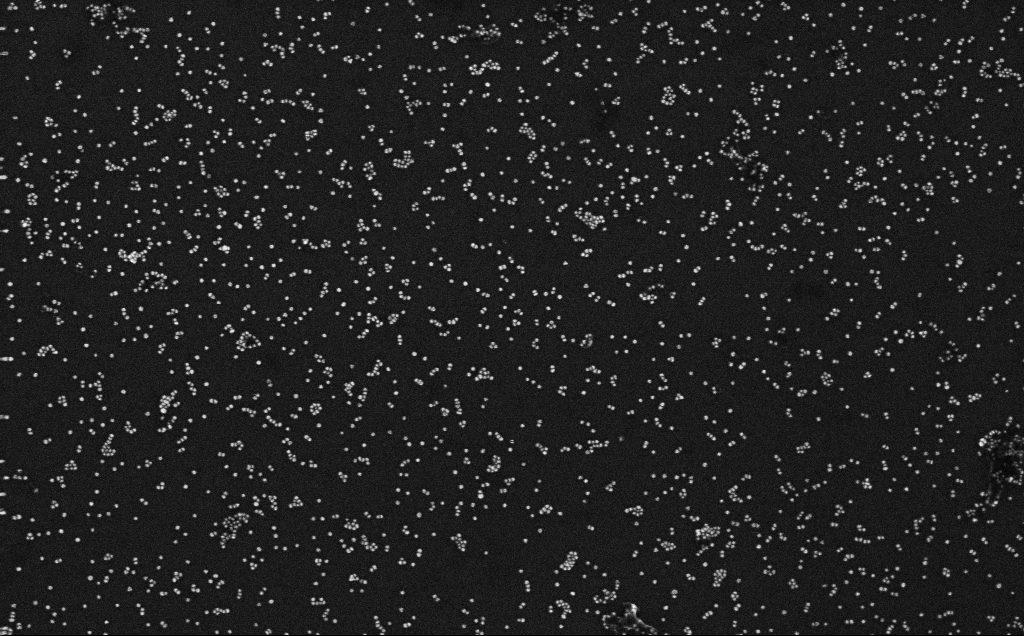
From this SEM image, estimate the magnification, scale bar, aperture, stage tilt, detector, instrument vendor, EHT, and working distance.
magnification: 100 K X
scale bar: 200 nm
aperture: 30 µm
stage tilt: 0°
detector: InLens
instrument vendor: Zeiss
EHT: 10 kV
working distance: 3.3 mm